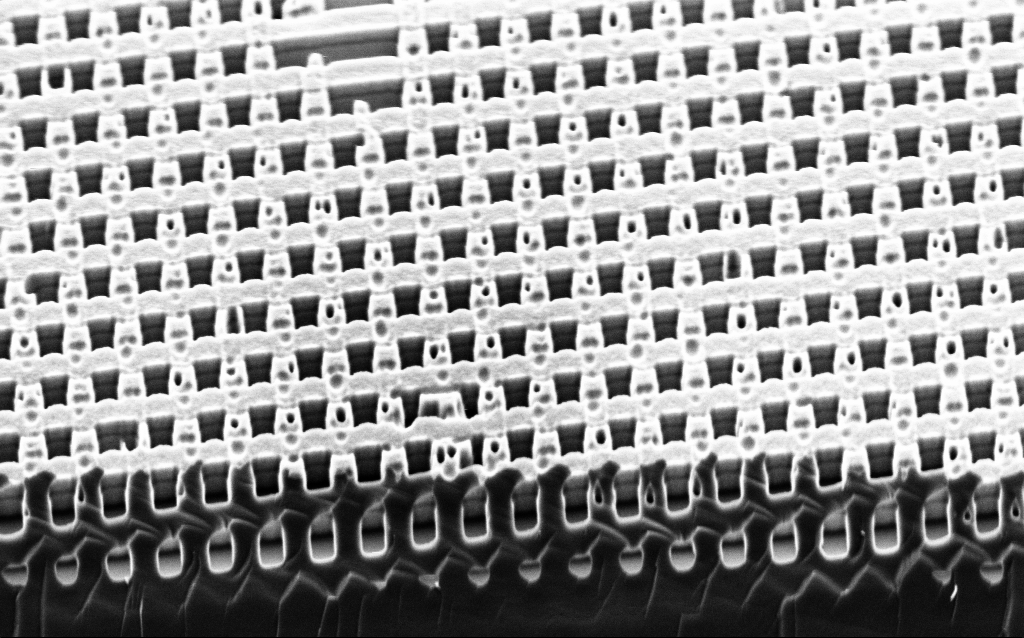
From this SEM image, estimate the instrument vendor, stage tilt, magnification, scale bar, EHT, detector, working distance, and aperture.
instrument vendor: Zeiss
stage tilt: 45°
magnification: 37.69 K X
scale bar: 1000 nm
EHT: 2 kV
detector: InLens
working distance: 3 mm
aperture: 30 µm